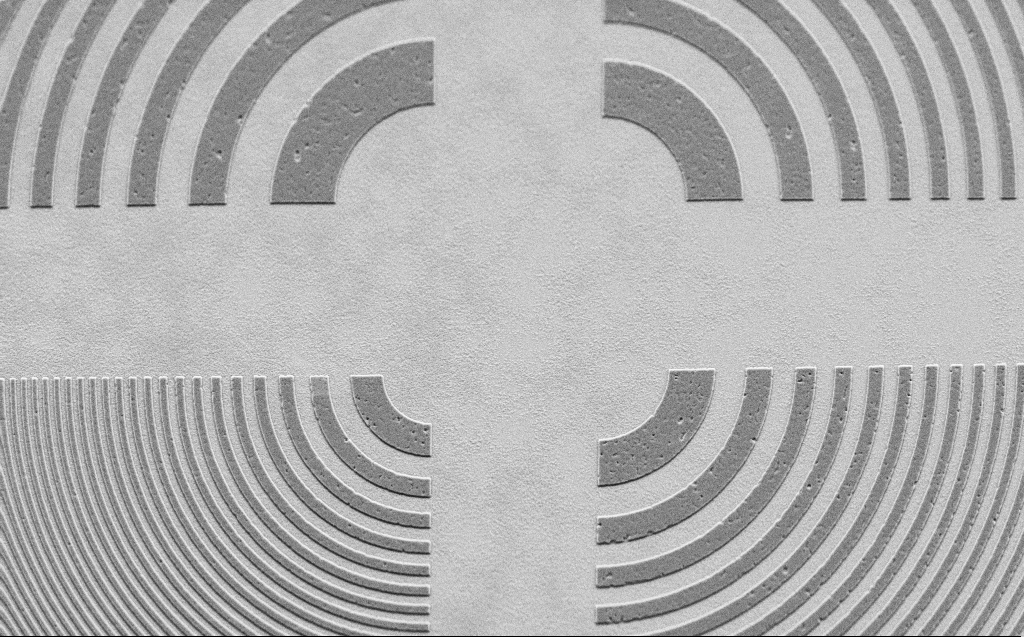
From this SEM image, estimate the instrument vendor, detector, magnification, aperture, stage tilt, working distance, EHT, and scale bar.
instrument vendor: Zeiss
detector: SE2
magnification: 6.24 K X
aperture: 30 µm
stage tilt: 44.1°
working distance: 6 mm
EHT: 2.5 kV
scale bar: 10000 nm